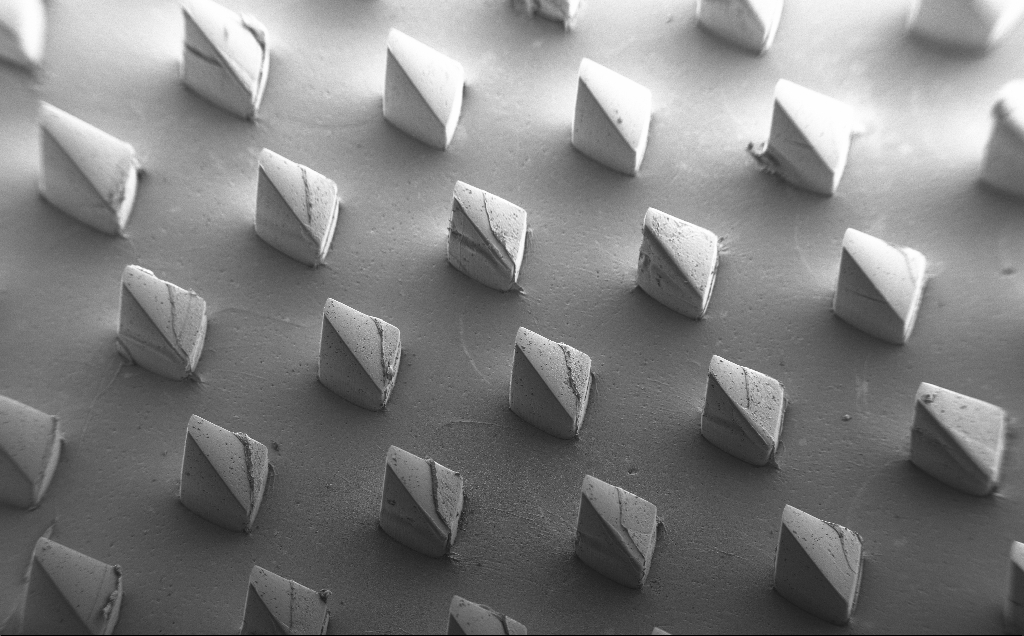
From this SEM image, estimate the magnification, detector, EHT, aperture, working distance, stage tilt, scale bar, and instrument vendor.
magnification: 0.077 K X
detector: SE2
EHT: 5 kV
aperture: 30 µm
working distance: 8 mm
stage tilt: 39°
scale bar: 200000 nm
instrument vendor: Zeiss